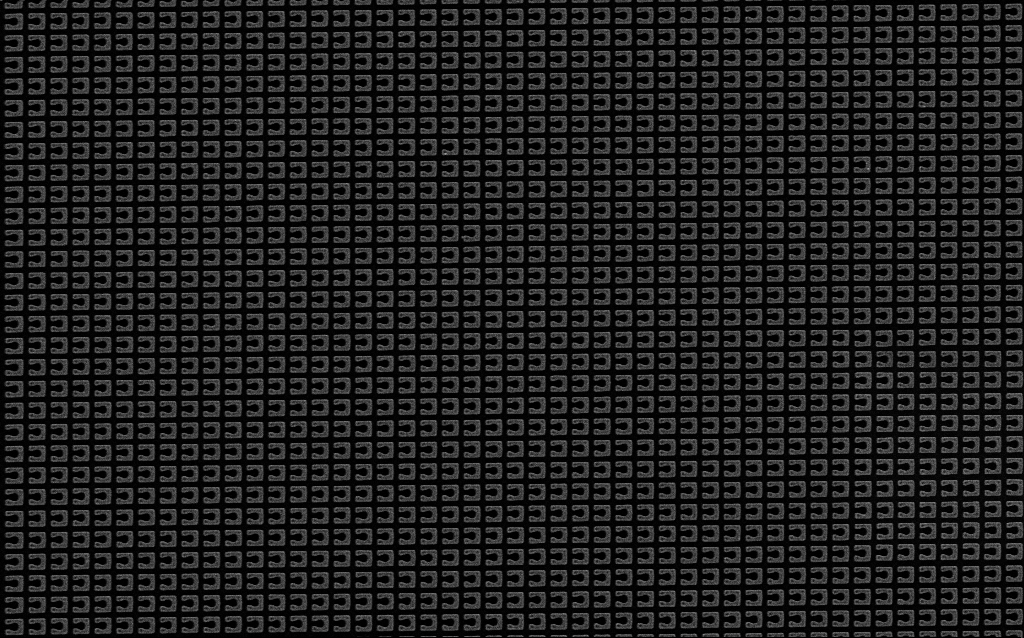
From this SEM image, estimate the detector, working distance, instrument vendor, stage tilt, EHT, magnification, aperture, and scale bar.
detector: SE2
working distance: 4.3 mm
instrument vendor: Zeiss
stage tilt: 0°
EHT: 3 kV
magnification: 17.34 K X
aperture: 30 µm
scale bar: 1000 nm